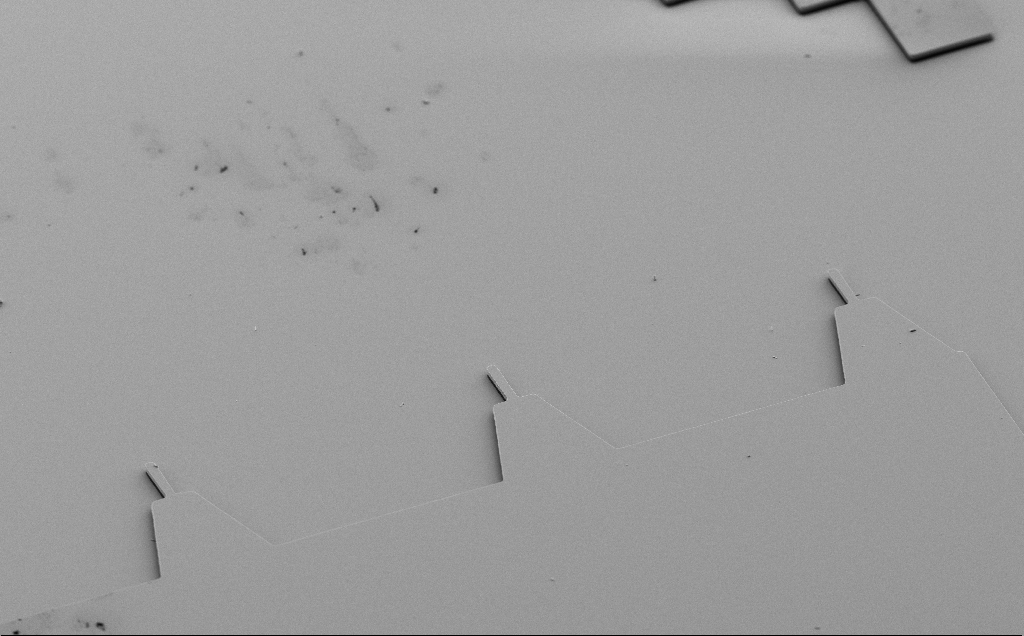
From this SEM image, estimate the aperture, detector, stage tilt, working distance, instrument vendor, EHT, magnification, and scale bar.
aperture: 30 µm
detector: SE2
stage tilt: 50°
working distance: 10 mm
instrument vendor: Zeiss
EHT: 5 kV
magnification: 0.855 K X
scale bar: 20000 nm